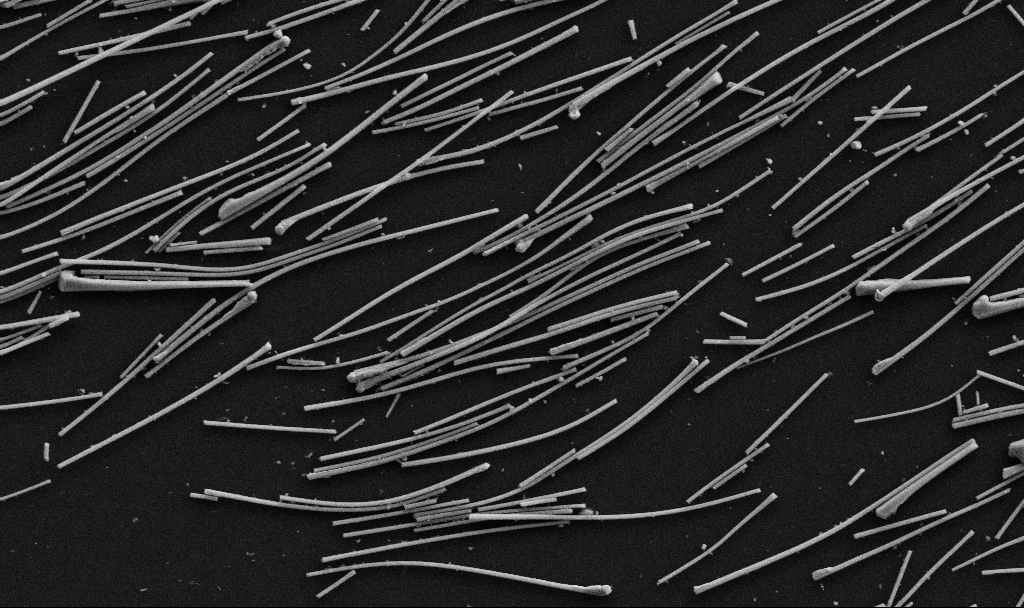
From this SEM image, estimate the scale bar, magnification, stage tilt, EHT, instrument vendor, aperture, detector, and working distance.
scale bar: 2000 nm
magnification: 18.28 K X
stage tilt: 0°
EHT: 5 kV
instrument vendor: Zeiss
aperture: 30 µm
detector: SE2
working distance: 6.7 mm